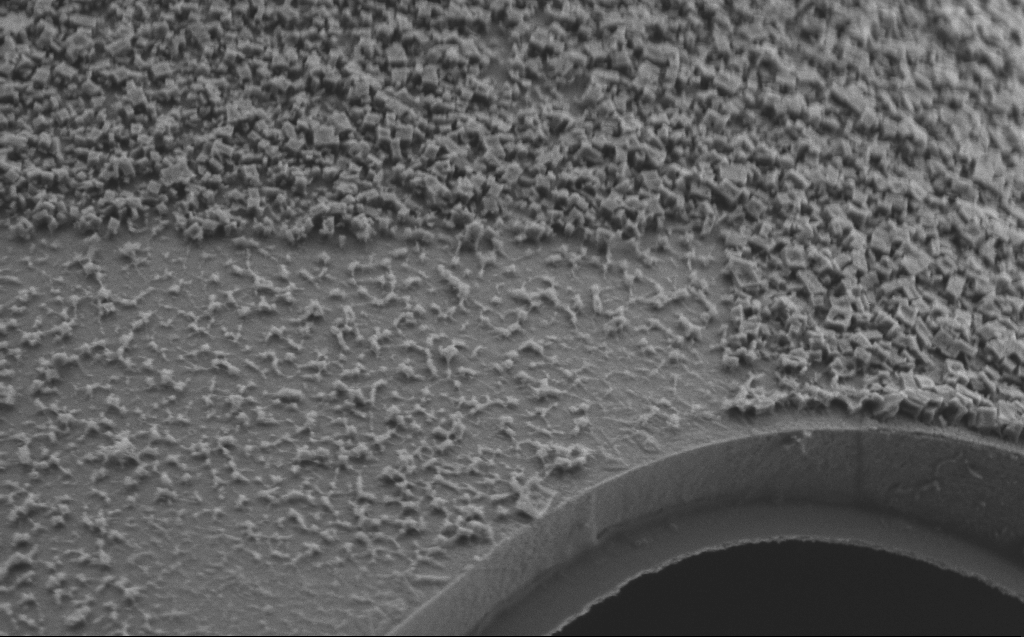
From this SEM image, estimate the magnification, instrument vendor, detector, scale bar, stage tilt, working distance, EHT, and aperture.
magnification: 26.84 K X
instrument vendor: Zeiss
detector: SE2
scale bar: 2000 nm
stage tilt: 45°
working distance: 4 mm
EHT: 2 kV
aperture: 30 µm